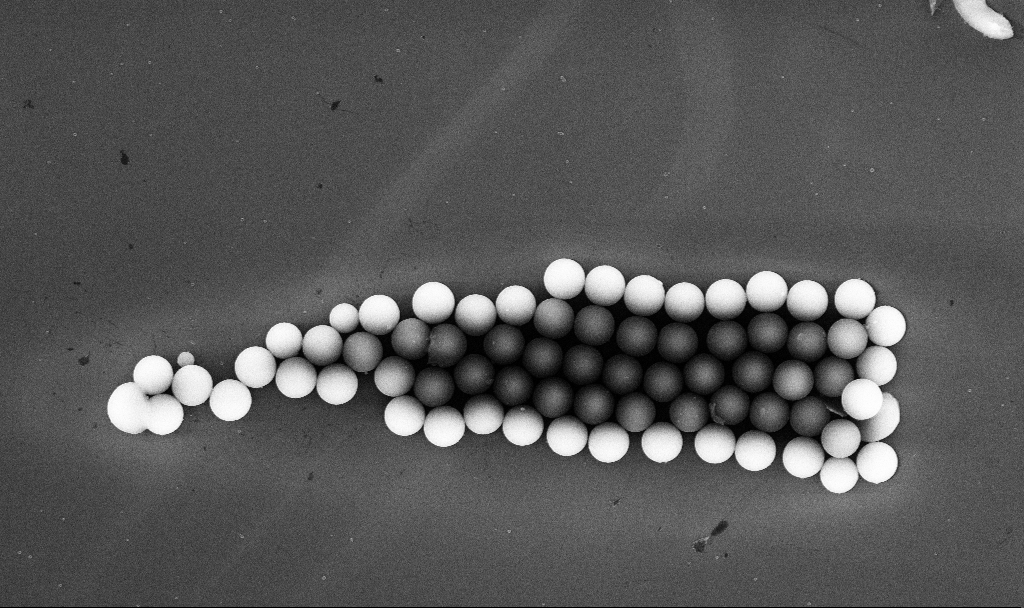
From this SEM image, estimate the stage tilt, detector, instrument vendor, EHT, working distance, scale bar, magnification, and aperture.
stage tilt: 0°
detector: InLens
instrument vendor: Zeiss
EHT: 10 kV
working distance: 5.2 mm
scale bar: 2000 nm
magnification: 7.29 K X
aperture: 30 µm